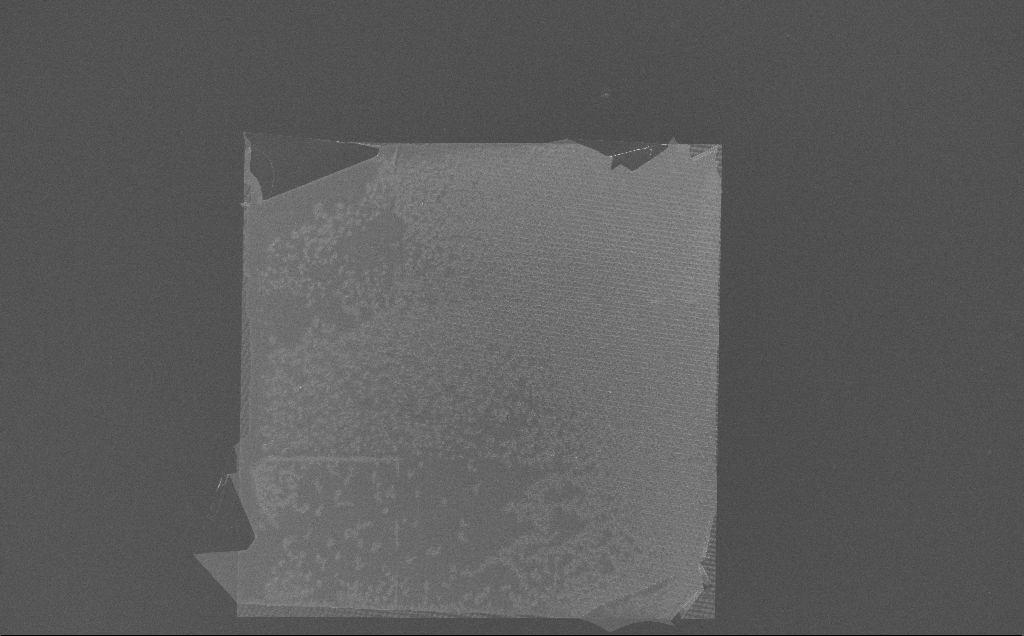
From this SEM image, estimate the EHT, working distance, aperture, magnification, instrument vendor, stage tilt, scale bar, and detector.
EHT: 10 kV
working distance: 7 mm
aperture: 30 µm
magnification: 0.238 K X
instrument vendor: Zeiss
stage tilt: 0°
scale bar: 100000 nm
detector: InLens